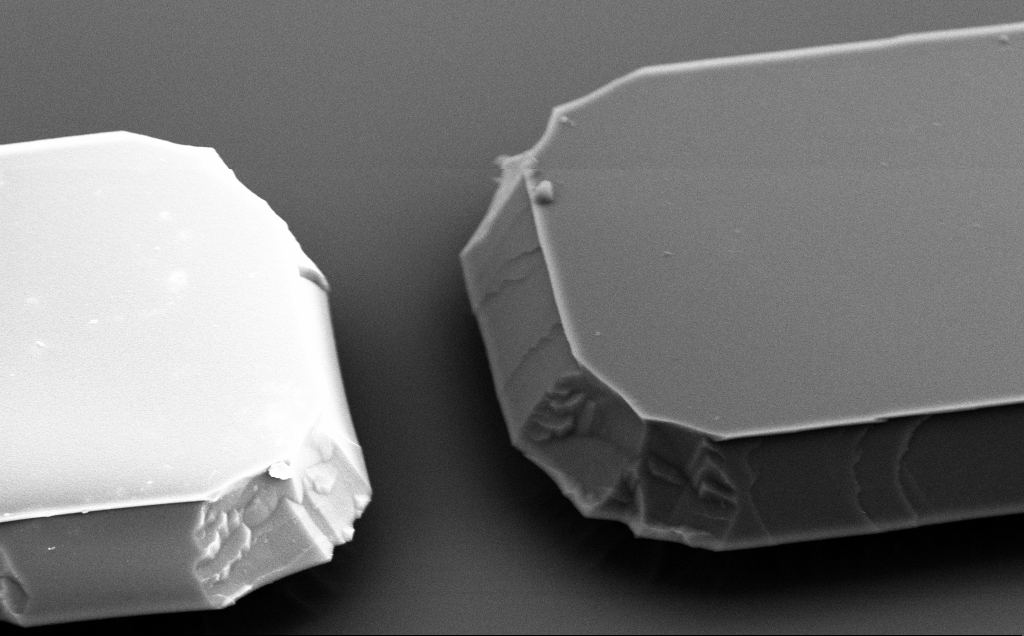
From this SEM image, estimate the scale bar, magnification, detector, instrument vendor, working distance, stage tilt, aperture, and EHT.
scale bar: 2000 nm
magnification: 14.31 K X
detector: SE2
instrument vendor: Zeiss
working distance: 10 mm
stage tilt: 50°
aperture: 30 µm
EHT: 5 kV